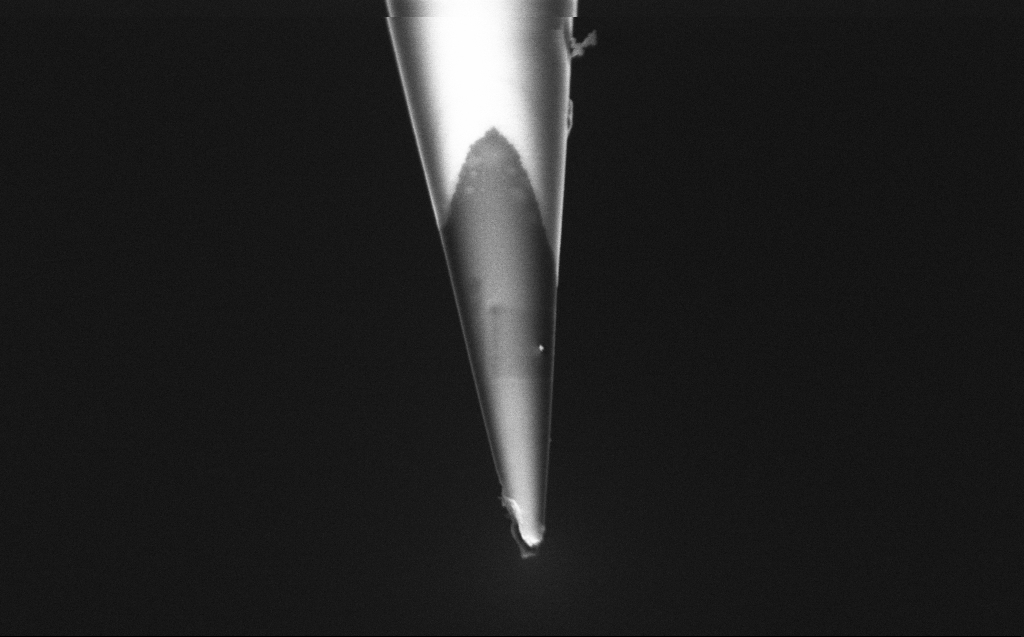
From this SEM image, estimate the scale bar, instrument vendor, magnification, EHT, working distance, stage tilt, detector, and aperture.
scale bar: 1000 nm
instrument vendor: Zeiss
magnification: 50 K X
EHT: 2 kV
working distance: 6 mm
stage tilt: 45°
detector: InLens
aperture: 30 µm